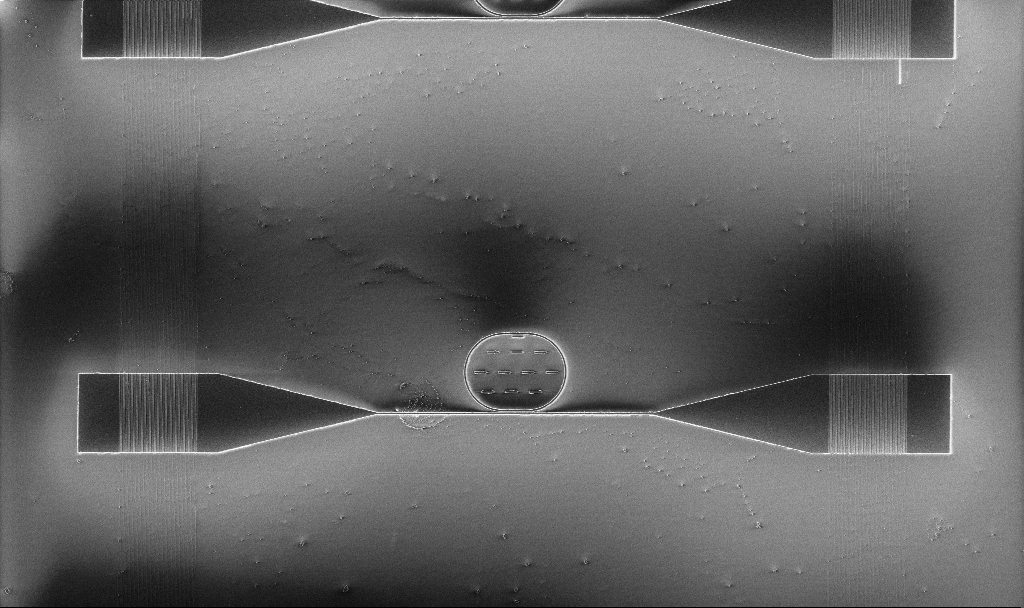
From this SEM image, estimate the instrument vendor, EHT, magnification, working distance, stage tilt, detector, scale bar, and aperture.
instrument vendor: Zeiss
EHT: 3 kV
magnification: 1.47 K X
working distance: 3 mm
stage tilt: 0°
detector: InLens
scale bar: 10000 nm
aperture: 30 µm